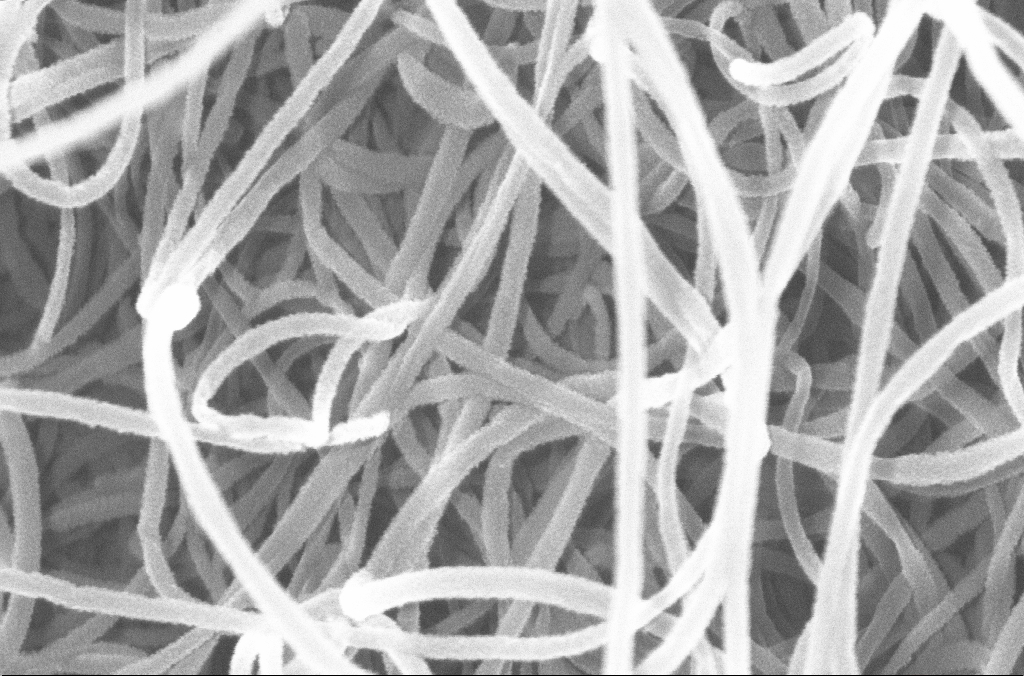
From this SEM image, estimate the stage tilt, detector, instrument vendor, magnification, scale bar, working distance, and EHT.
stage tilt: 0°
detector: InLens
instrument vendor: Zeiss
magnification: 200 K X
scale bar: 100 nm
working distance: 4.2 mm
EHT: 20 kV